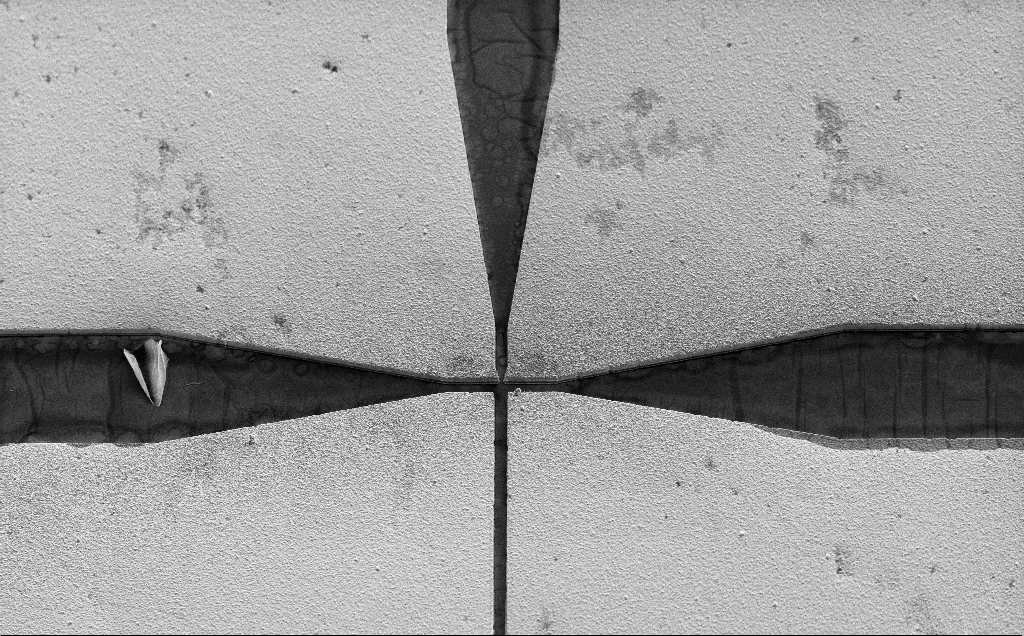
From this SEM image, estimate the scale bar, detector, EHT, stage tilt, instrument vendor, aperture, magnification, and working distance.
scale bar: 100000 nm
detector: SE2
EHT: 10 kV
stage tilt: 45°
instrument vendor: Zeiss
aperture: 30 µm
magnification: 0.525 K X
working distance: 15 mm